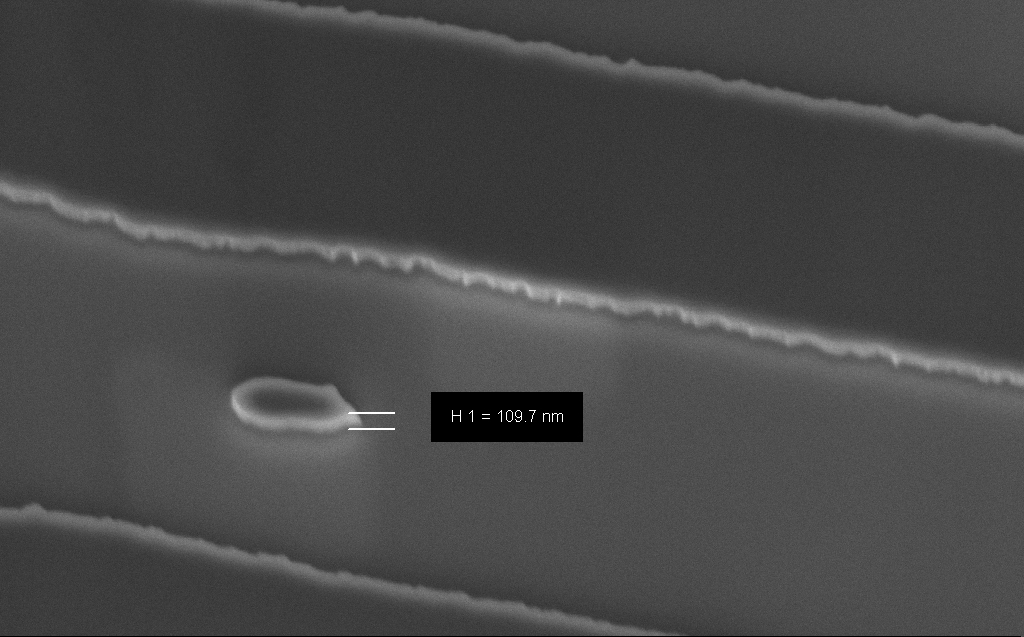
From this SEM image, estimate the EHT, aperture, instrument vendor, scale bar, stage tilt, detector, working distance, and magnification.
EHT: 3 kV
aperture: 30 µm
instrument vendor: Zeiss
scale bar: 1000 nm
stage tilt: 45°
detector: InLens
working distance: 9 mm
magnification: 53.53 K X